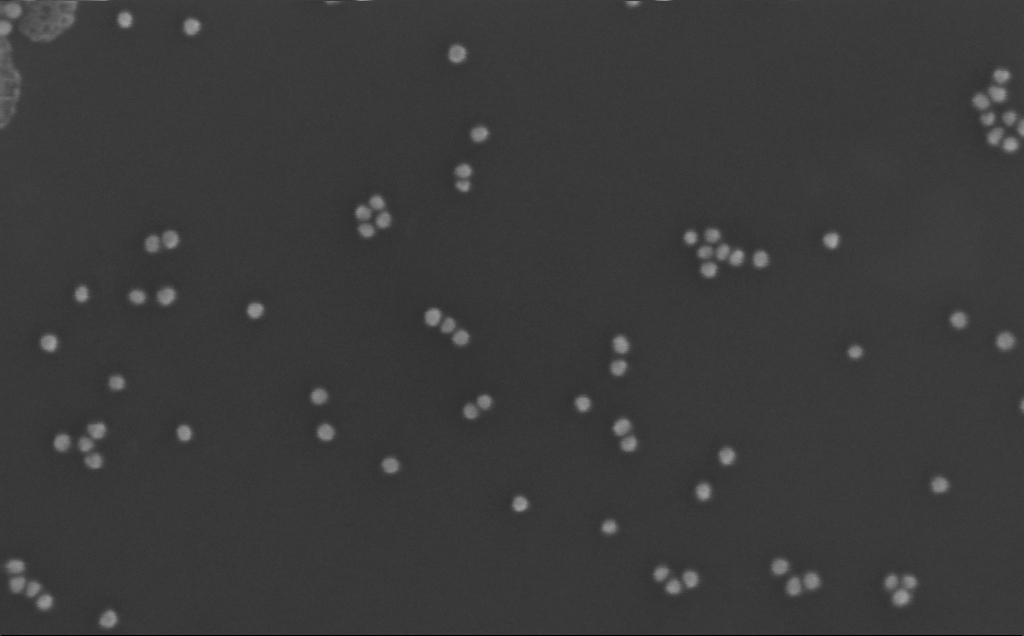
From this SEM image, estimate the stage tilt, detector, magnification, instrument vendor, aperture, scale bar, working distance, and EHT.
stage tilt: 0°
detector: InLens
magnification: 354.97 K X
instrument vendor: Zeiss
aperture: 30 µm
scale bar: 200 nm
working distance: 3 mm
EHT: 10 kV